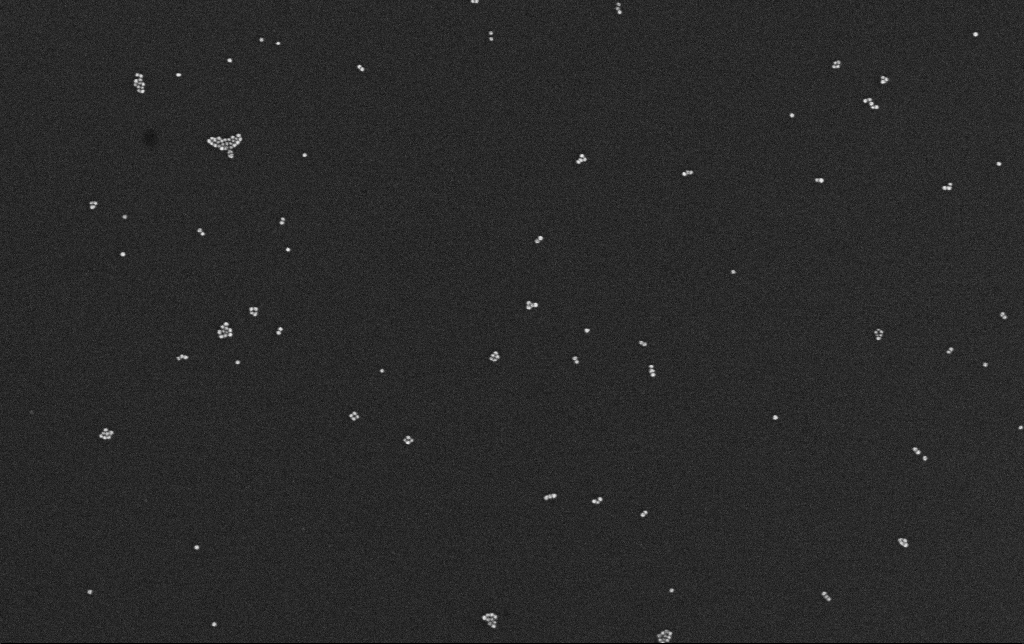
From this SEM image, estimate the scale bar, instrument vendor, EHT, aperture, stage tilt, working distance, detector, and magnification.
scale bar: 200 nm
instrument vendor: Zeiss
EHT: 10 kV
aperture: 30 µm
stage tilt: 0°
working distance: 3.1 mm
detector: InLens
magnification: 100 K X